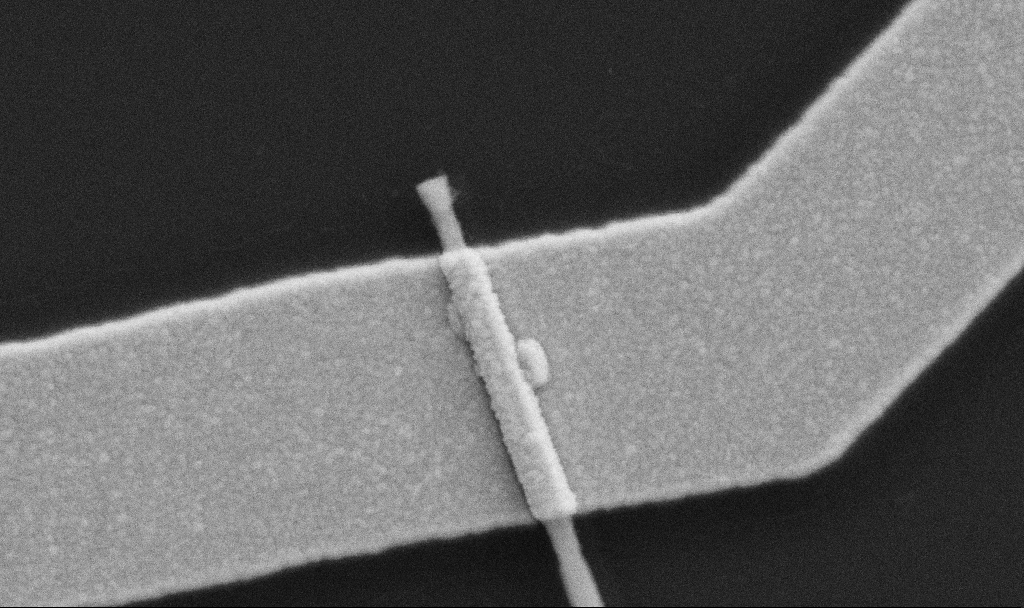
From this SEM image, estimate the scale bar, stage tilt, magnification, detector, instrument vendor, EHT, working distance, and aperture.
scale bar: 200 nm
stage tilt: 0°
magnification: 100 K X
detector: SE2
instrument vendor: Zeiss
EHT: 5 kV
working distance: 10.7 mm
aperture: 30 µm